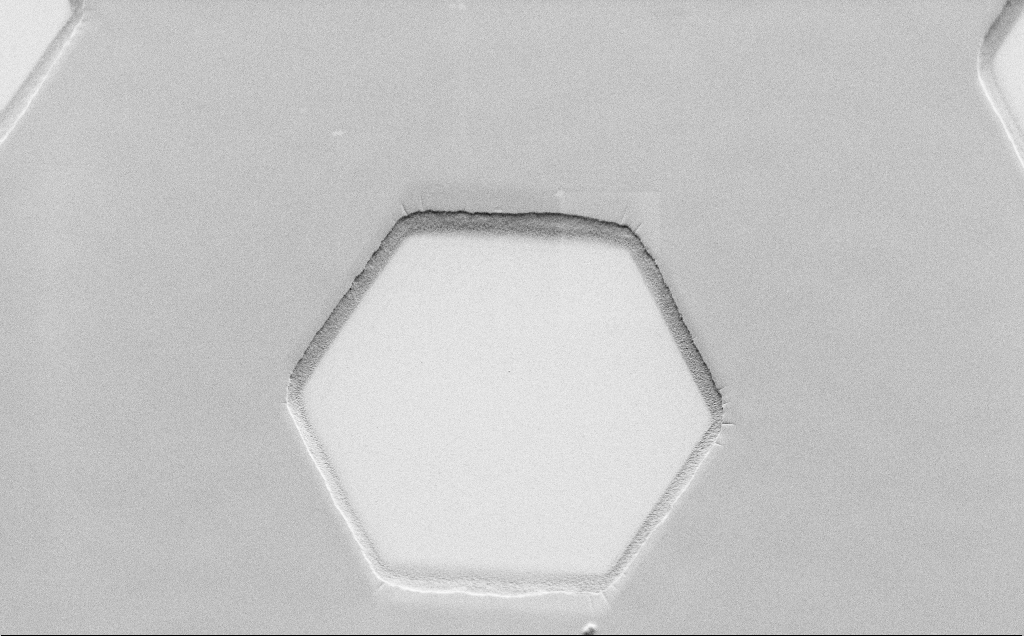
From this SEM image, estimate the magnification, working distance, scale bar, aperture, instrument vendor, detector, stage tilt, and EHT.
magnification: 2.85 K X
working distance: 6 mm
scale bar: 10000 nm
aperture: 30 µm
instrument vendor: Zeiss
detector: SE2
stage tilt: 45°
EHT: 1.5 kV